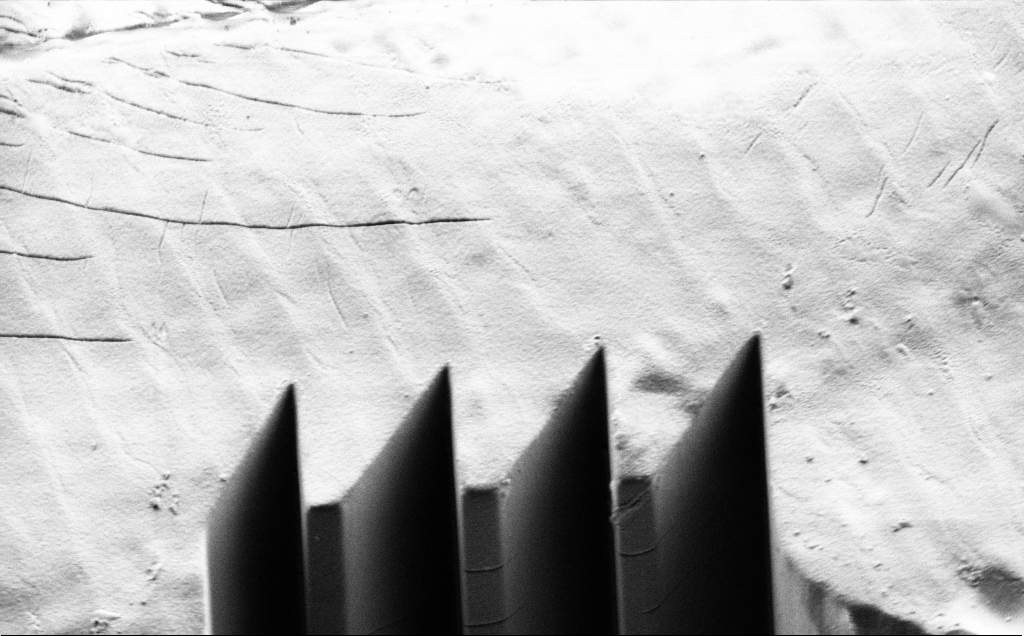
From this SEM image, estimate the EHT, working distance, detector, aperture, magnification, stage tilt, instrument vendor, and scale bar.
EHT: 1.2 kV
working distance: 7 mm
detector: SE2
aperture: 30 µm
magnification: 4.93 K X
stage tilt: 45°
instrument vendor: Zeiss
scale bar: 10000 nm